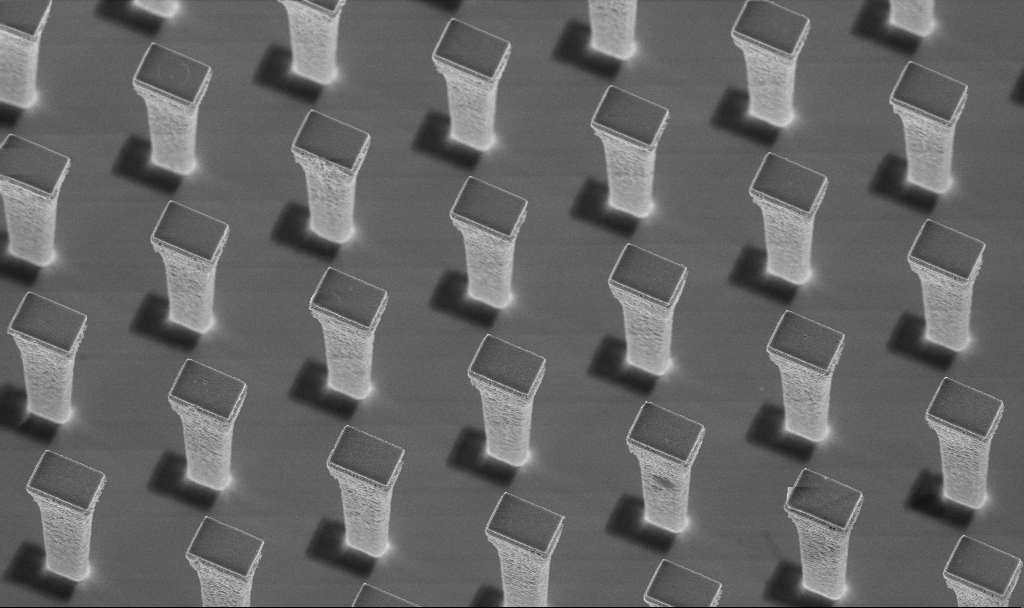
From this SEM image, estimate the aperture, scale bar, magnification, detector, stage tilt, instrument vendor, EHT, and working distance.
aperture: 30 µm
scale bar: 10000 nm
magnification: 5.34 K X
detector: InLens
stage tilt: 20°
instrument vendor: Zeiss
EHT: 5 kV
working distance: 4.3 mm